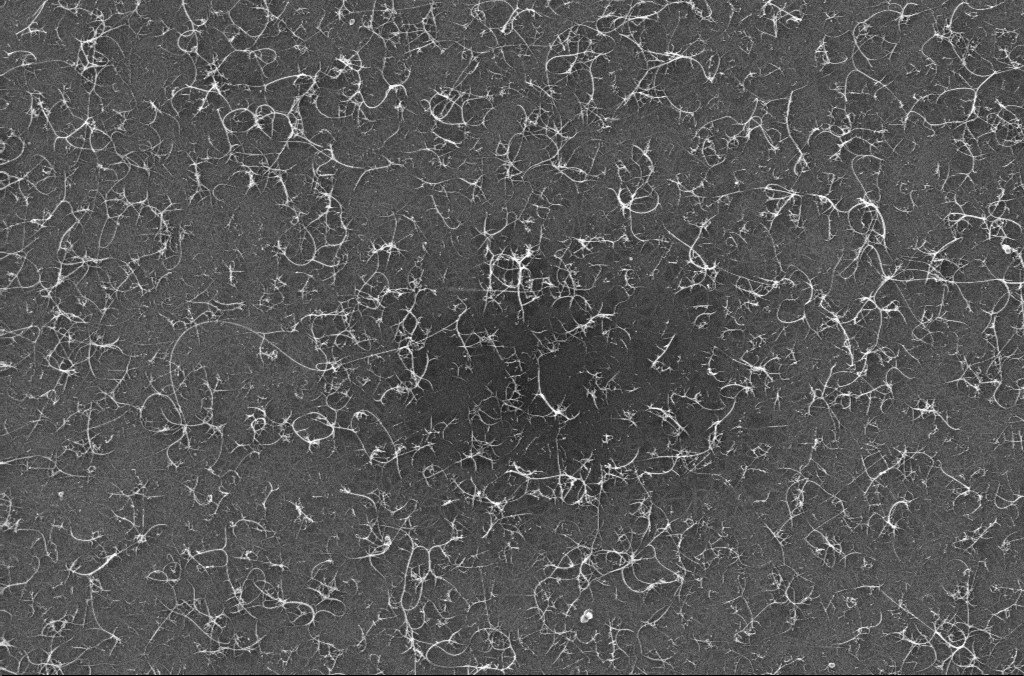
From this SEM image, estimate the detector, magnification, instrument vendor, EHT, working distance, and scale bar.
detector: InLens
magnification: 53.78 K X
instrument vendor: Zeiss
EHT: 10 kV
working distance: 3.2 mm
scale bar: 1000 nm